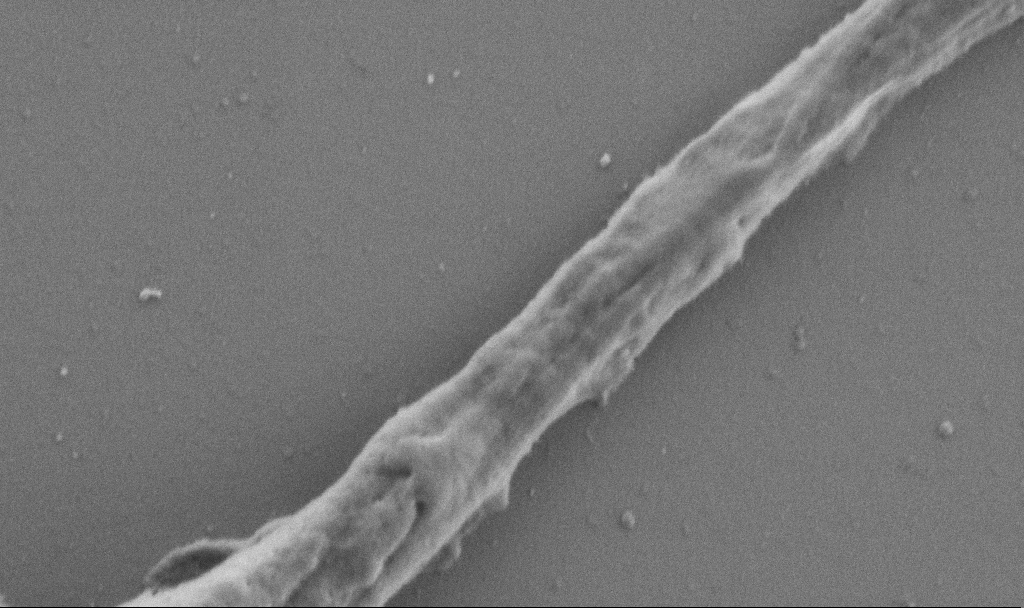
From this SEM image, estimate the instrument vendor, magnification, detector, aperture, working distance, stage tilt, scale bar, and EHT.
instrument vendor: Zeiss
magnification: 50 K X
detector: SE2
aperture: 30 µm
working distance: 6.9 mm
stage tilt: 0°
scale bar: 1000 nm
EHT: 1 kV